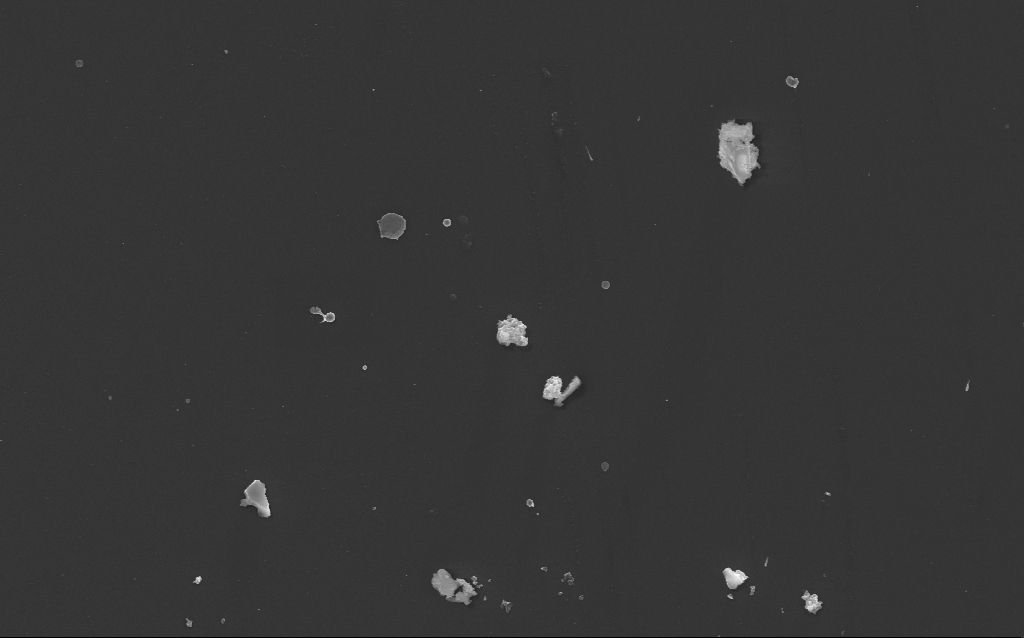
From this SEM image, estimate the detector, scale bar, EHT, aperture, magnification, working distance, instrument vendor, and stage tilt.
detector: InLens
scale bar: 10000 nm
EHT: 10 kV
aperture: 30 µm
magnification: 6.12 K X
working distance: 3 mm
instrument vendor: Zeiss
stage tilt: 0°